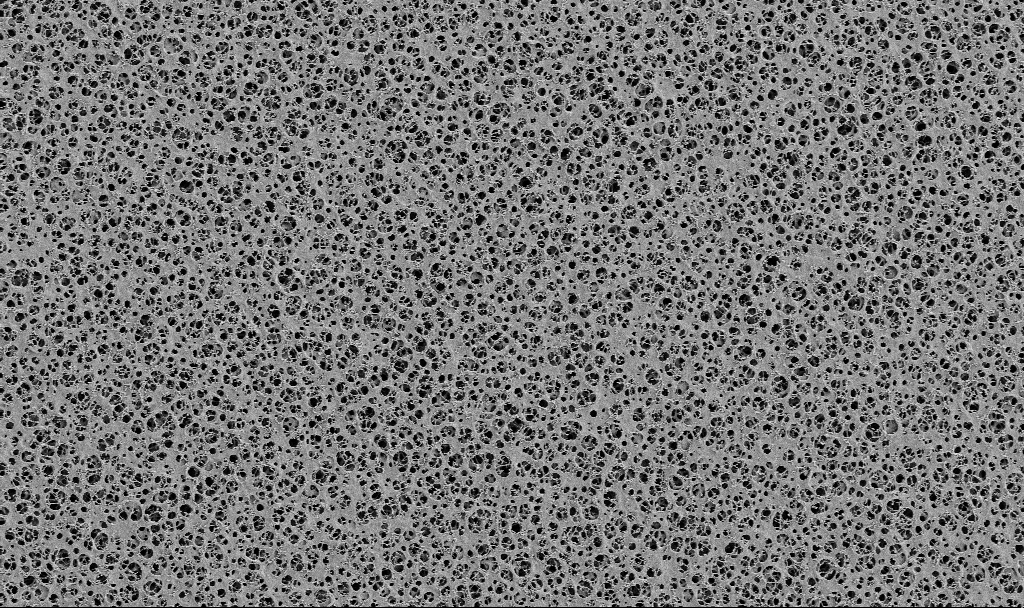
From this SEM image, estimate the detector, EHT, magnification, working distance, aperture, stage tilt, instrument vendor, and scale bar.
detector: SE2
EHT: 2 kV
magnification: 2 K X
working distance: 3.4 mm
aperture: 30 µm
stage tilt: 0°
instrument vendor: Zeiss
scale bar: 10000 nm